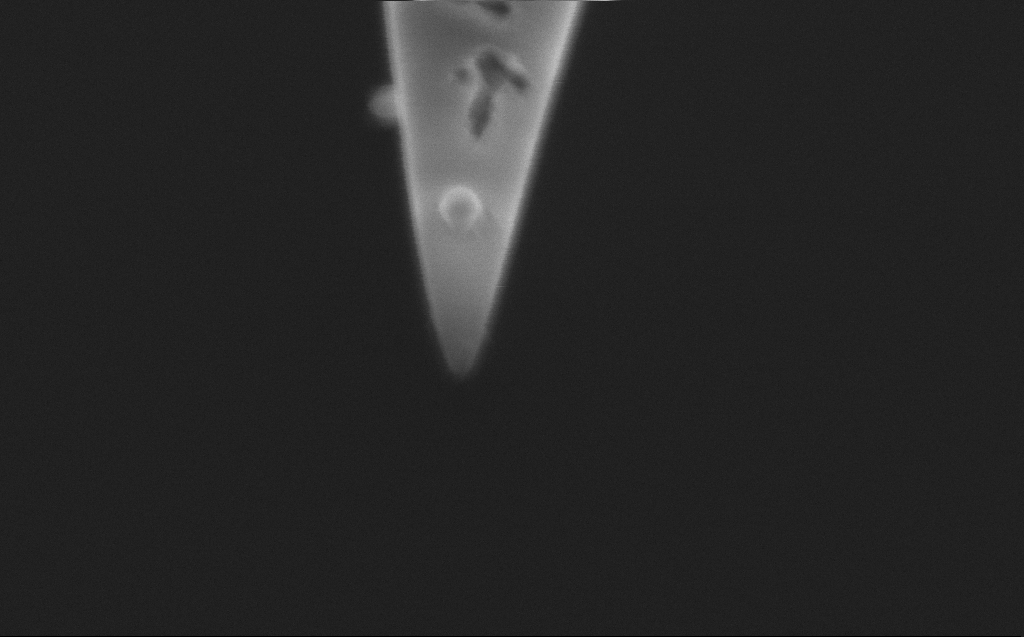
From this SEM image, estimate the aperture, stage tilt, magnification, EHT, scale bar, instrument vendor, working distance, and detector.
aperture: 20 µm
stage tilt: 45.1°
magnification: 138.72 K X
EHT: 2 kV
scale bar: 100 nm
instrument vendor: Zeiss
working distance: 4 mm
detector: InLens